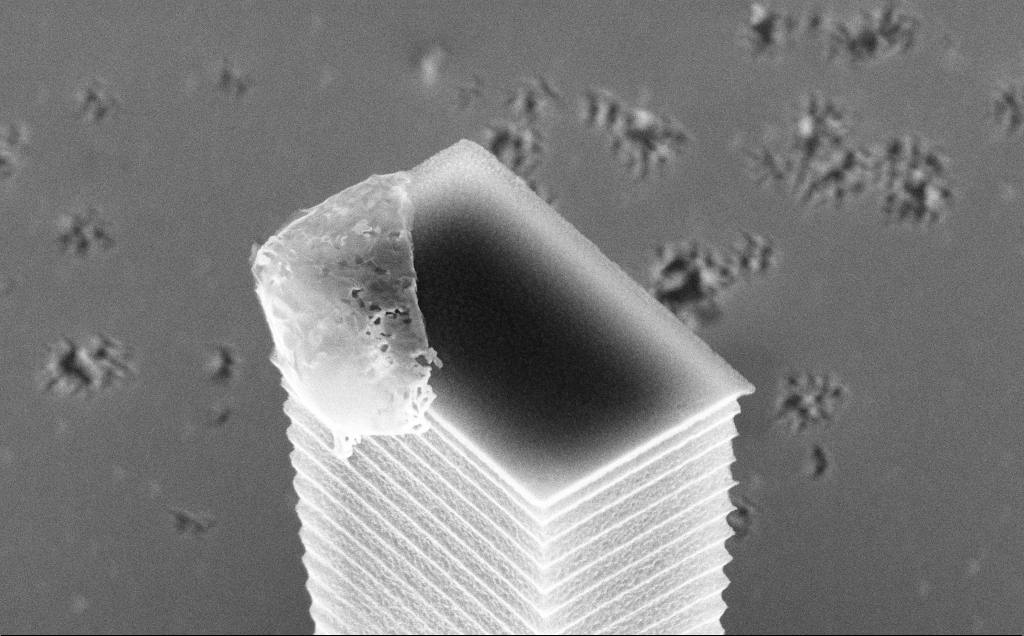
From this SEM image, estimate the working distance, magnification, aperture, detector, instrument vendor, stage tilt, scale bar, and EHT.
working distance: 7 mm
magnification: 34.44 K X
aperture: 30 µm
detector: InLens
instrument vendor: Zeiss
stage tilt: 45°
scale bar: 1000 nm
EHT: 10 kV